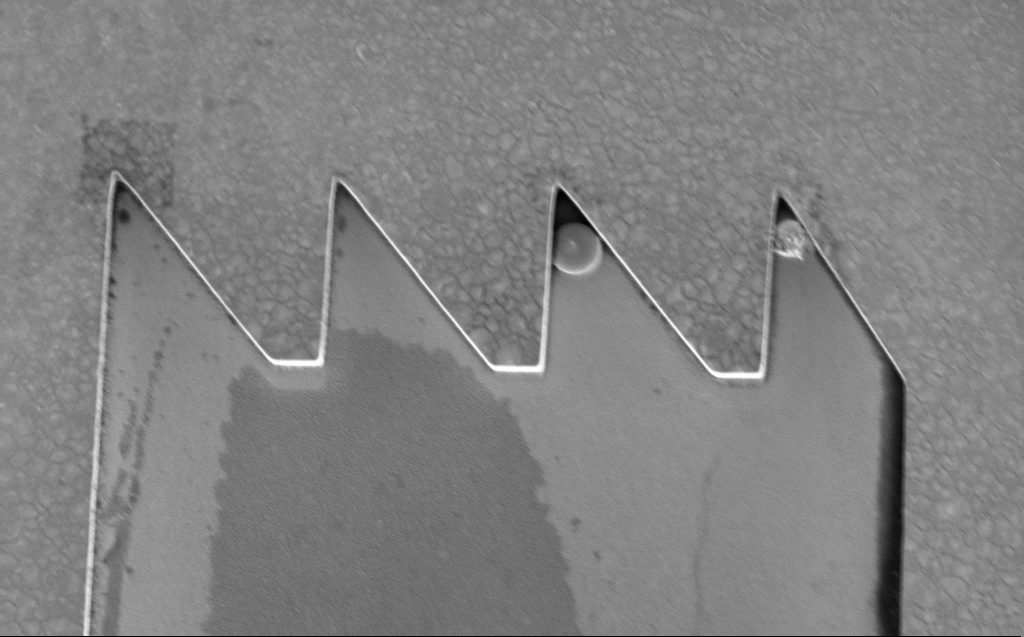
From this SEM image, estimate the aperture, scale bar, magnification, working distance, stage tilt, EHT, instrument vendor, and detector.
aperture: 120 µm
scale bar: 10000 nm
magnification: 7.06 K X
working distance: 8 mm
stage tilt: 0°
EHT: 3 kV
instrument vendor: Zeiss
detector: InLens